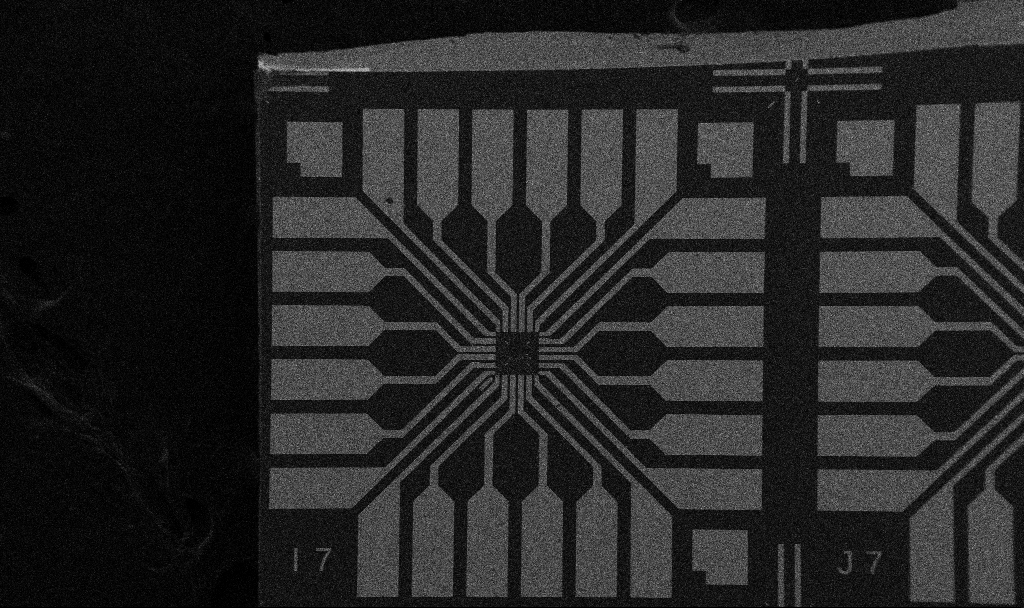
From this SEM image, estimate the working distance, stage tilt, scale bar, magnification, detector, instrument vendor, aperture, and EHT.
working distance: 10.7 mm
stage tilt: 0°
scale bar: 200000 nm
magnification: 0.1 K X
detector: SE2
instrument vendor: Zeiss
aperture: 30 µm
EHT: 5 kV